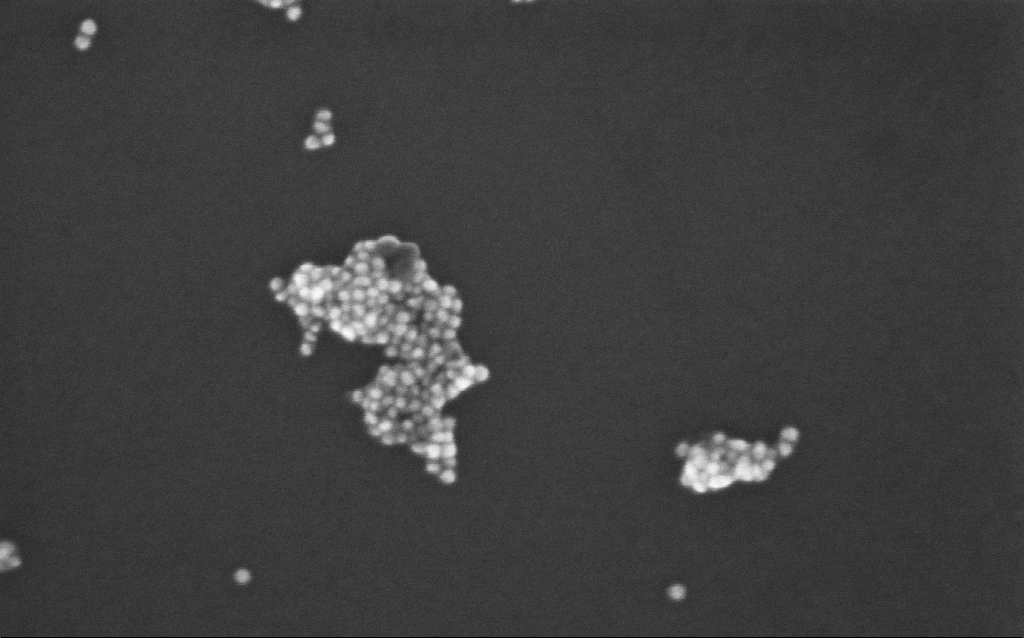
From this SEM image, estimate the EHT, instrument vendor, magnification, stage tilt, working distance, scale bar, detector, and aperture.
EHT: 10 kV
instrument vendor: Zeiss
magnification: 315.23 K X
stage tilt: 0°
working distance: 7 mm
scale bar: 200 nm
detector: InLens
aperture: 30 µm